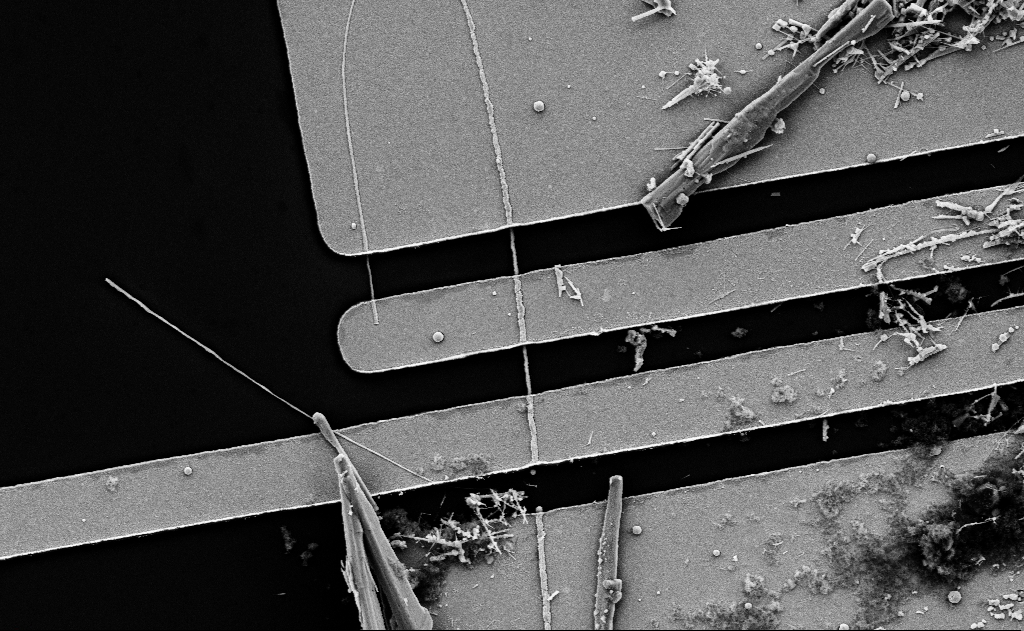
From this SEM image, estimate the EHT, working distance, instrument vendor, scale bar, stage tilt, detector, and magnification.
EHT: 5 kV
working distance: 18 mm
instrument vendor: Zeiss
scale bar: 2000 nm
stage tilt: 0°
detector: SE2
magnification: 11.1 K X